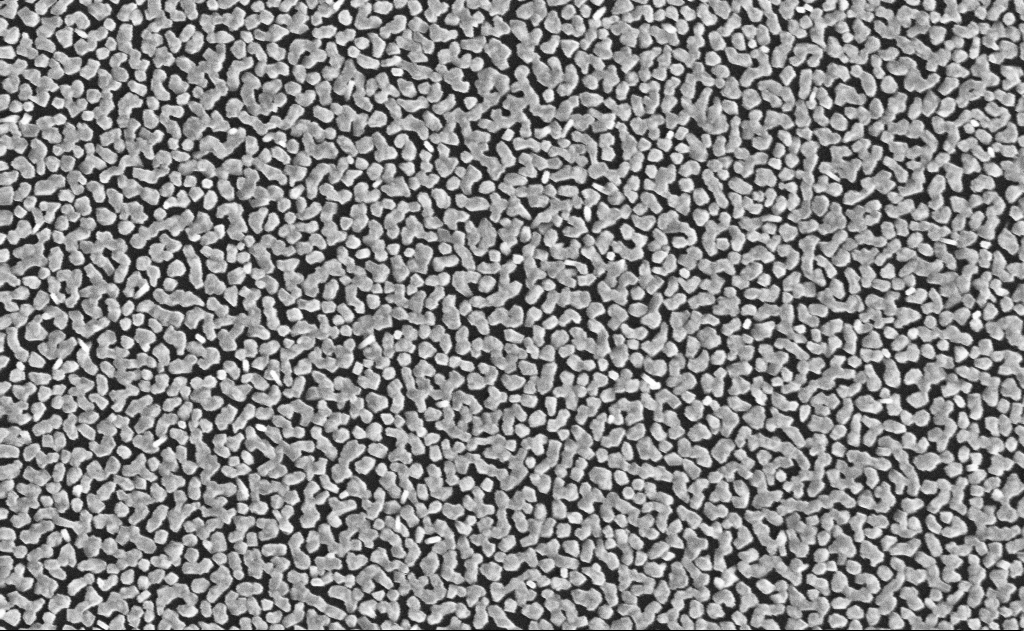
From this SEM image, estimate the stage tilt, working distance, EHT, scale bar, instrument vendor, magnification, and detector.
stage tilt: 0°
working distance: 16 mm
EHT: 10 kV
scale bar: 1000 nm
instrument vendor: Zeiss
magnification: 40 K X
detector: InLens